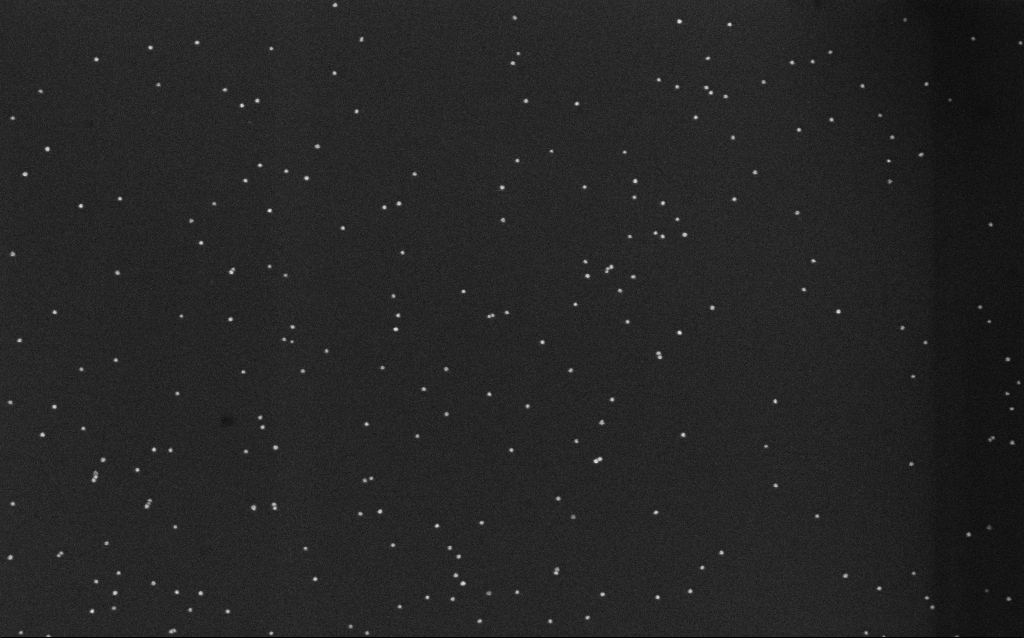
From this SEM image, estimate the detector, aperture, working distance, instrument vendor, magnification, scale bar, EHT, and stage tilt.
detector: InLens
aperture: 30 µm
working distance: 6.5 mm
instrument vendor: Zeiss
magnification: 100 K X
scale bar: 200 nm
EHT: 10 kV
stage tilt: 0°